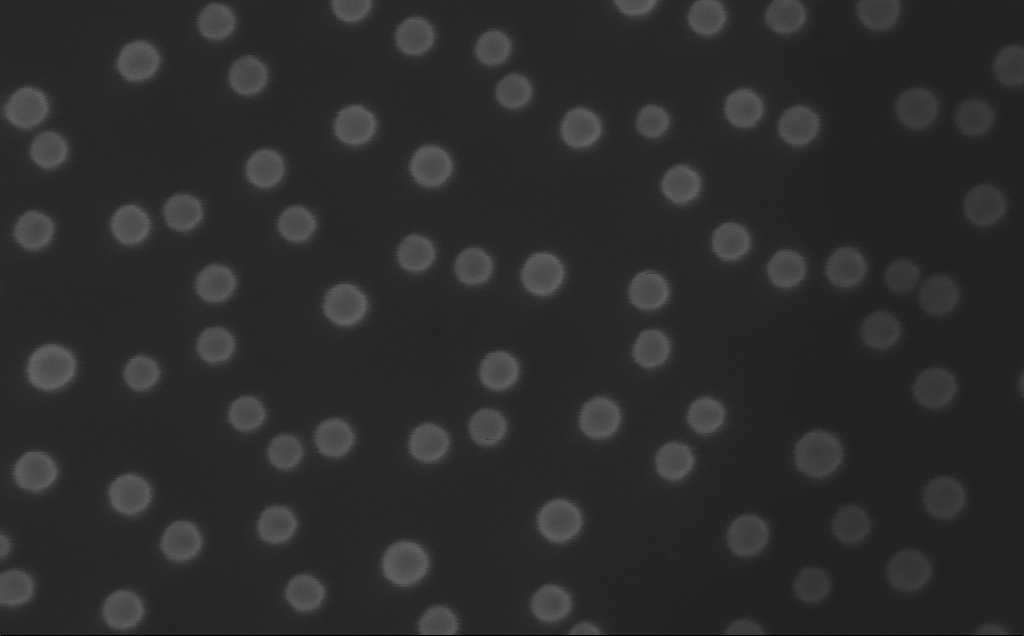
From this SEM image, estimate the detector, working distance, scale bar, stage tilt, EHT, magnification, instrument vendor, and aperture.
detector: InLens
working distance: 4 mm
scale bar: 100 nm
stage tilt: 0°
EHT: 10 kV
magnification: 500 K X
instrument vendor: Zeiss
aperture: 30 µm